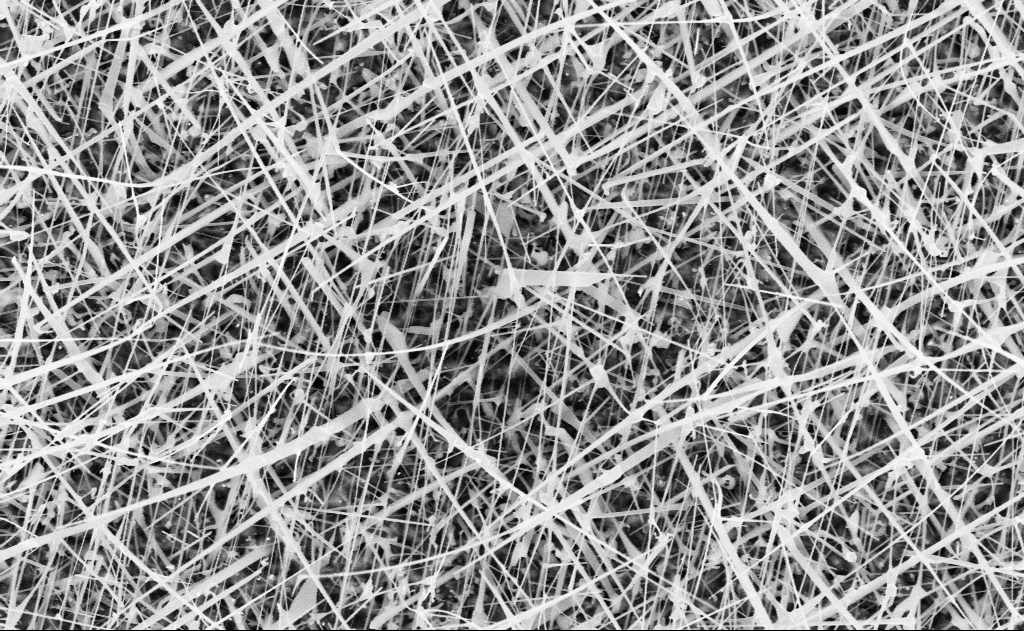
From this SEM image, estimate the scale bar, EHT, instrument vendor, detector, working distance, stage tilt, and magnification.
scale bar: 1000 nm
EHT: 10 kV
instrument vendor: Zeiss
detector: InLens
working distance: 20 mm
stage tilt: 0°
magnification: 20 K X